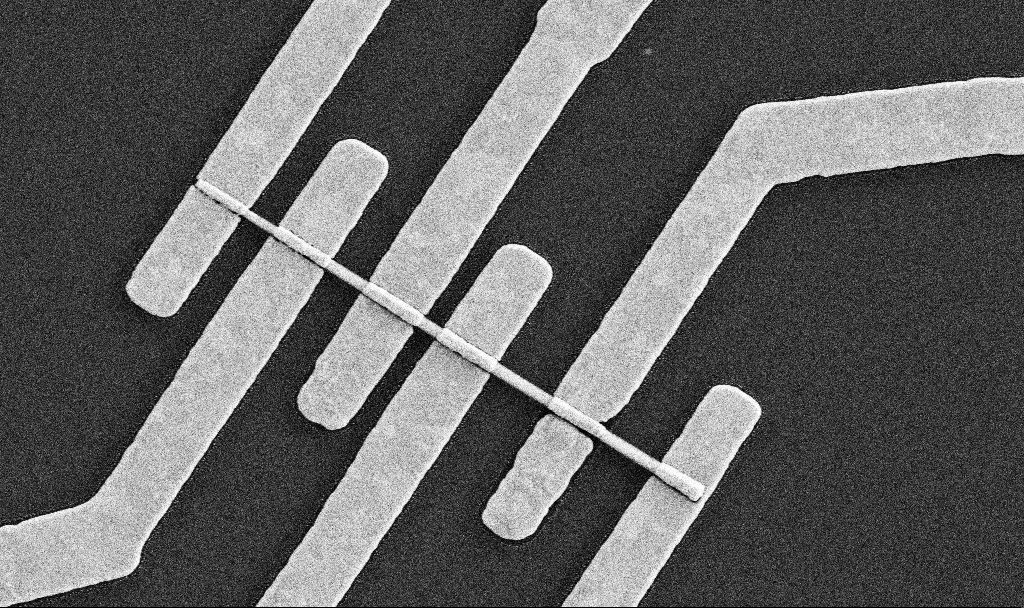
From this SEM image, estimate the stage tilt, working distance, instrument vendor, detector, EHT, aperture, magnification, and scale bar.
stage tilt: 0°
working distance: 7.6 mm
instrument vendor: Zeiss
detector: SE2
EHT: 5 kV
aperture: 30 µm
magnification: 34.58 K X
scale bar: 2000 nm